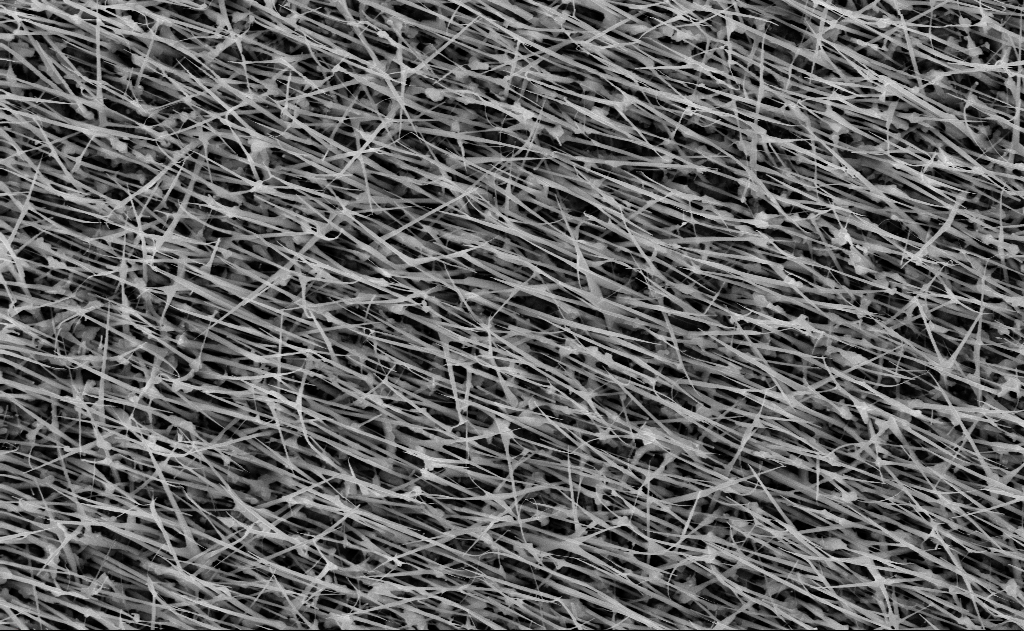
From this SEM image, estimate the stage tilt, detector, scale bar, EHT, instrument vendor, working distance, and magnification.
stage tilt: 0°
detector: InLens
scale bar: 2000 nm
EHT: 10 kV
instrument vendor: Zeiss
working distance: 15 mm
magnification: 20 K X